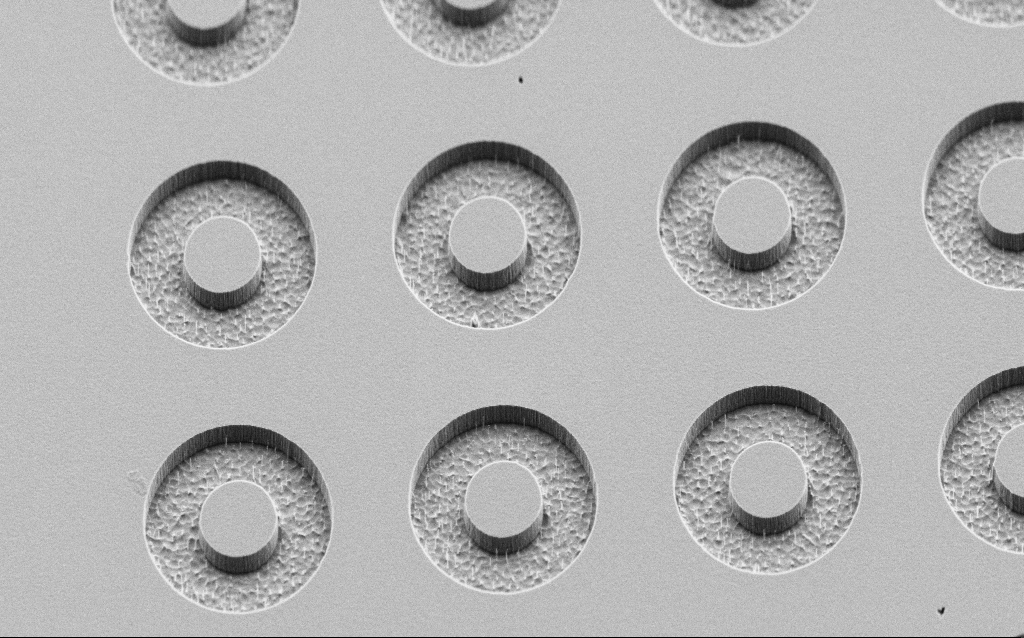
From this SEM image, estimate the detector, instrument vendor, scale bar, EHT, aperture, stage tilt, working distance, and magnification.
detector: SE2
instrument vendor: Zeiss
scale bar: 2000 nm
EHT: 2 kV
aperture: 30 µm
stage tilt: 45°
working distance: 6 mm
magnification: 9.79 K X